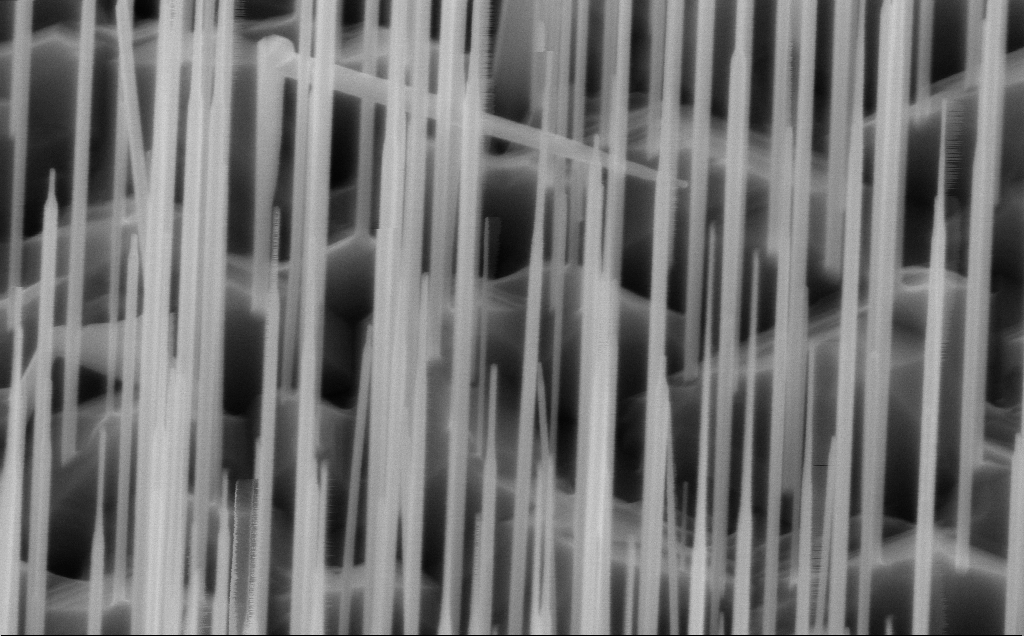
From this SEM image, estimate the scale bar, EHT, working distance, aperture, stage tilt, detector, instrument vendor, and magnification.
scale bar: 200 nm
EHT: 10 kV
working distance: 5 mm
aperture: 30 µm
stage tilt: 45°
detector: InLens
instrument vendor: Zeiss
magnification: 80 K X